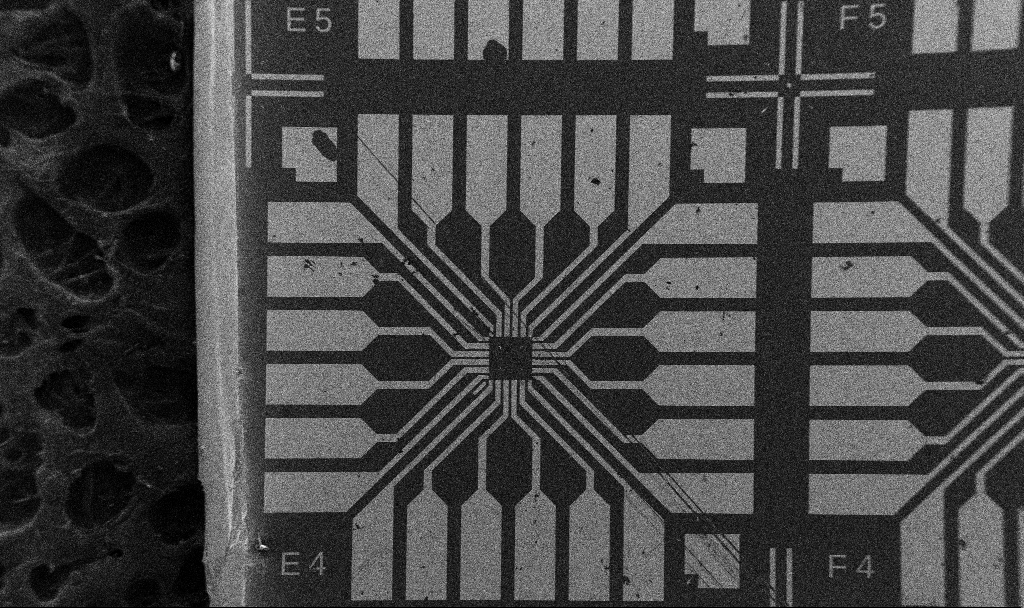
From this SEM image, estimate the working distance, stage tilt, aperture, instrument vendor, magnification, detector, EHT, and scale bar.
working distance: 9 mm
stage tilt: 0°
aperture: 30 µm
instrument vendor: Zeiss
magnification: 0.1 K X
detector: SE2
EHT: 5 kV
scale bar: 200000 nm